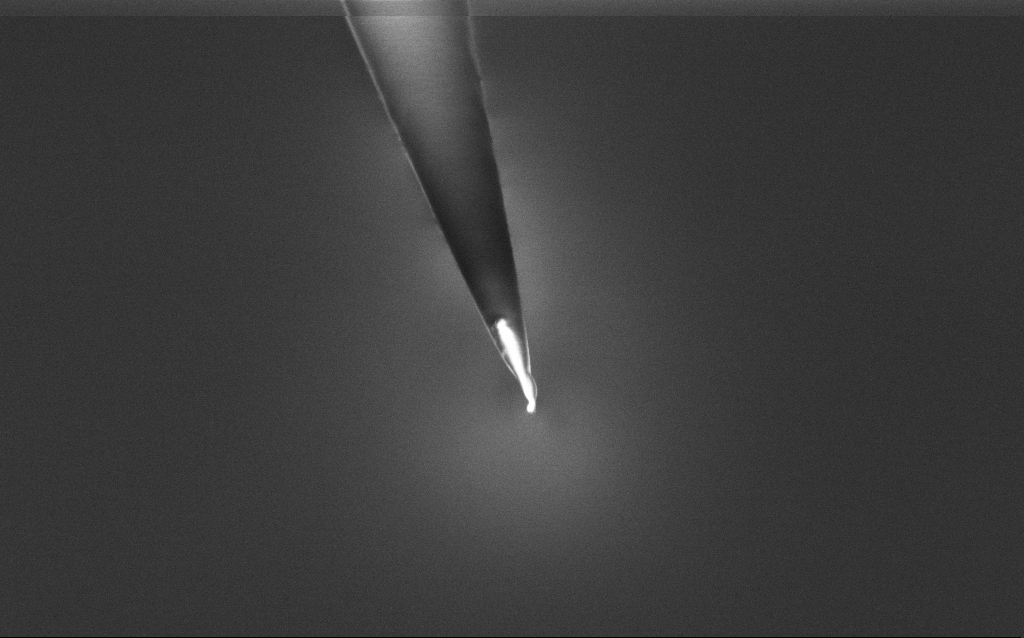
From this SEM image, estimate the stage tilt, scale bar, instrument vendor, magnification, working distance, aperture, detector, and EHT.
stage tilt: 45°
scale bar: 2000 nm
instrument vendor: Zeiss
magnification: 25 K X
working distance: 6 mm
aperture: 30 µm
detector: InLens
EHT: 1 kV